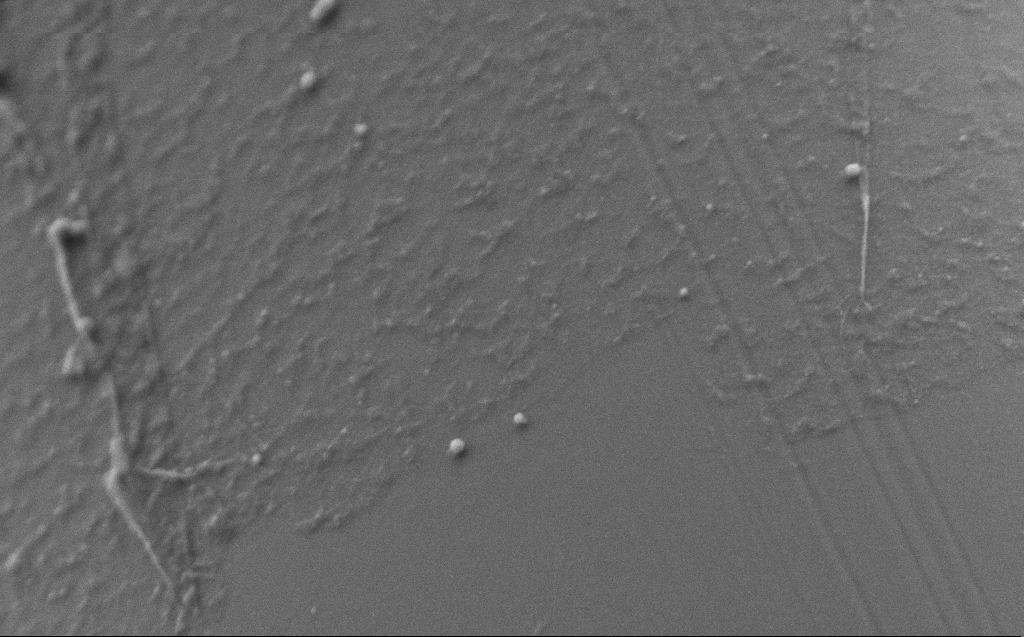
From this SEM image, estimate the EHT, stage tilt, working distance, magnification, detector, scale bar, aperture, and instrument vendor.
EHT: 2 kV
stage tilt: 45°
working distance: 3 mm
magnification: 50 K X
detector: SE2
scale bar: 1000 nm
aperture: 30 µm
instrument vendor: Zeiss